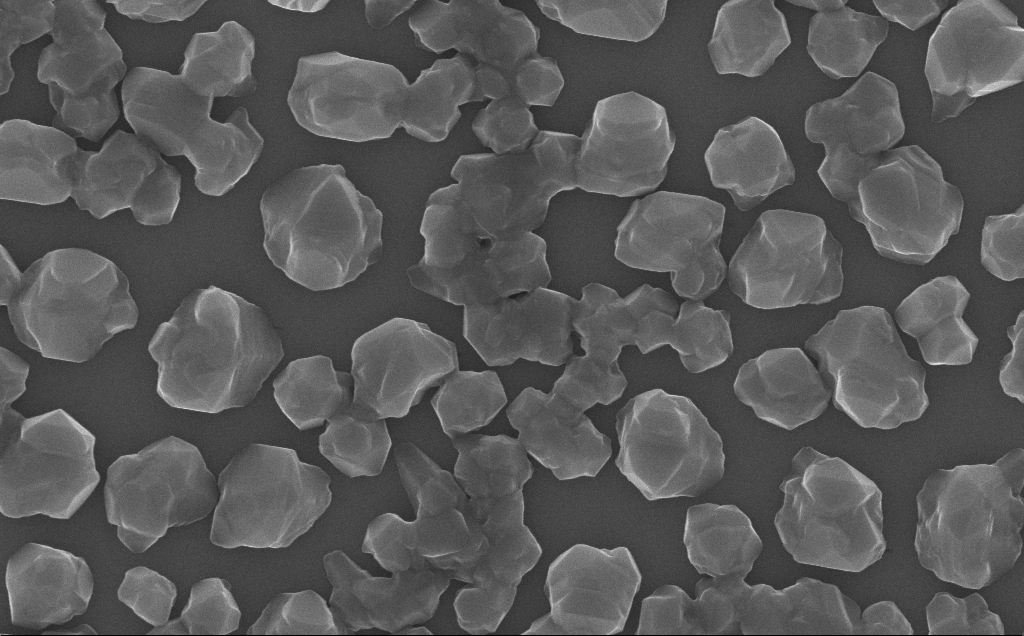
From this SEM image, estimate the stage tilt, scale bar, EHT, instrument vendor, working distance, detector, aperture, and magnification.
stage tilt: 0°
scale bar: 1000 nm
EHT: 10 kV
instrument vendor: Zeiss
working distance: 7 mm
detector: InLens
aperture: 30 µm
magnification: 67.46 K X